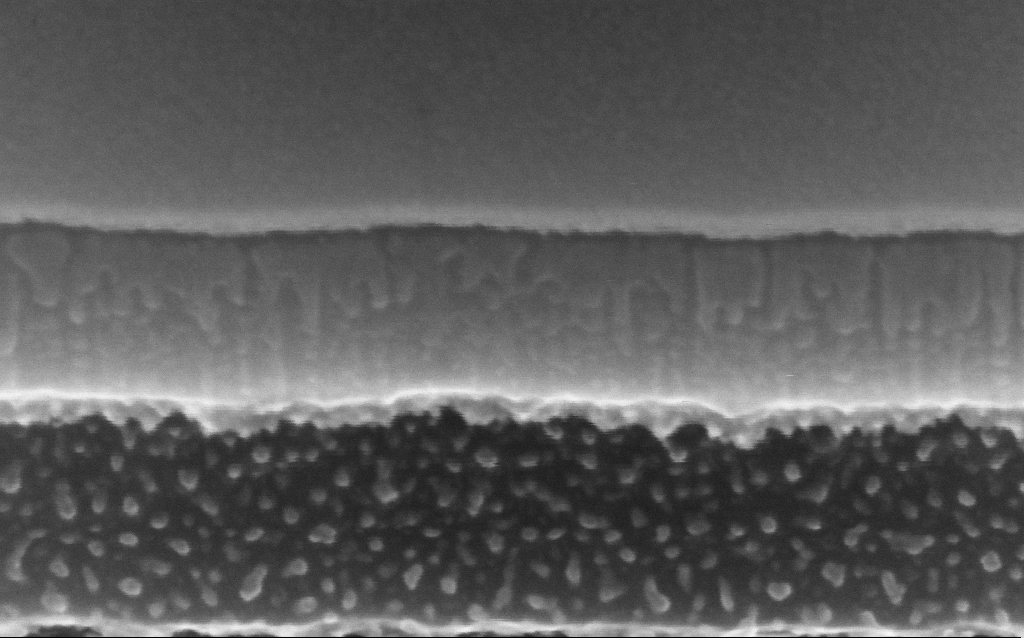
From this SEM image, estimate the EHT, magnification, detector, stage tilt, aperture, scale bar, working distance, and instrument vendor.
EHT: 10 kV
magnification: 461.66 K X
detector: InLens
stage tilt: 45°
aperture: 30 µm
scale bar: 100 nm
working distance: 4.8 mm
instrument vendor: Zeiss